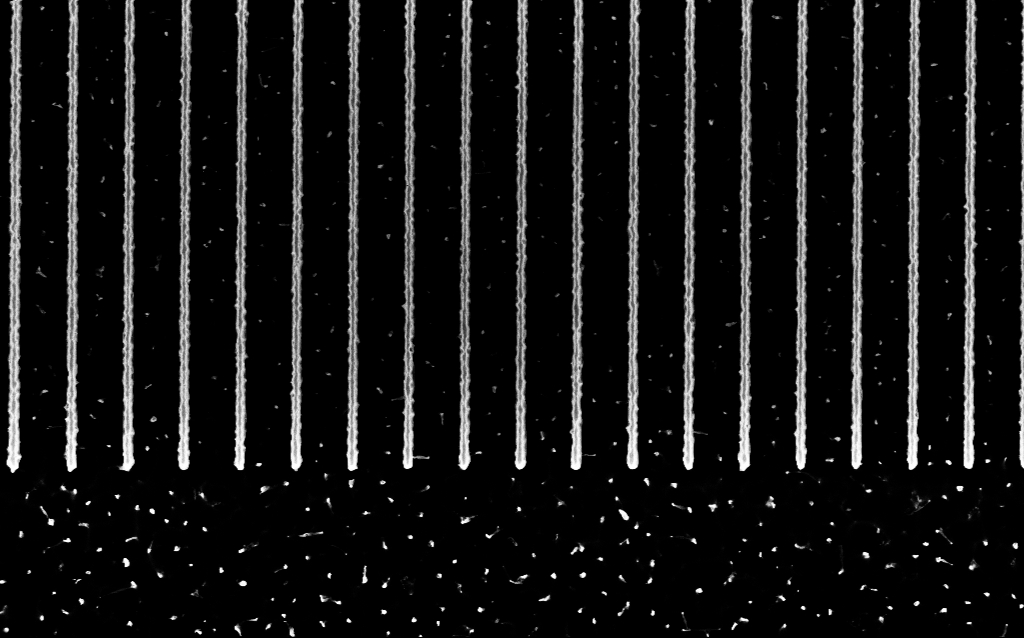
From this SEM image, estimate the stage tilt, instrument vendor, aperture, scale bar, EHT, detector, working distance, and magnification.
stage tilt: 0°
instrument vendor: Zeiss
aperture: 30 µm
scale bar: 1000 nm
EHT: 3 kV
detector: InLens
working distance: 5 mm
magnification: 41.46 K X